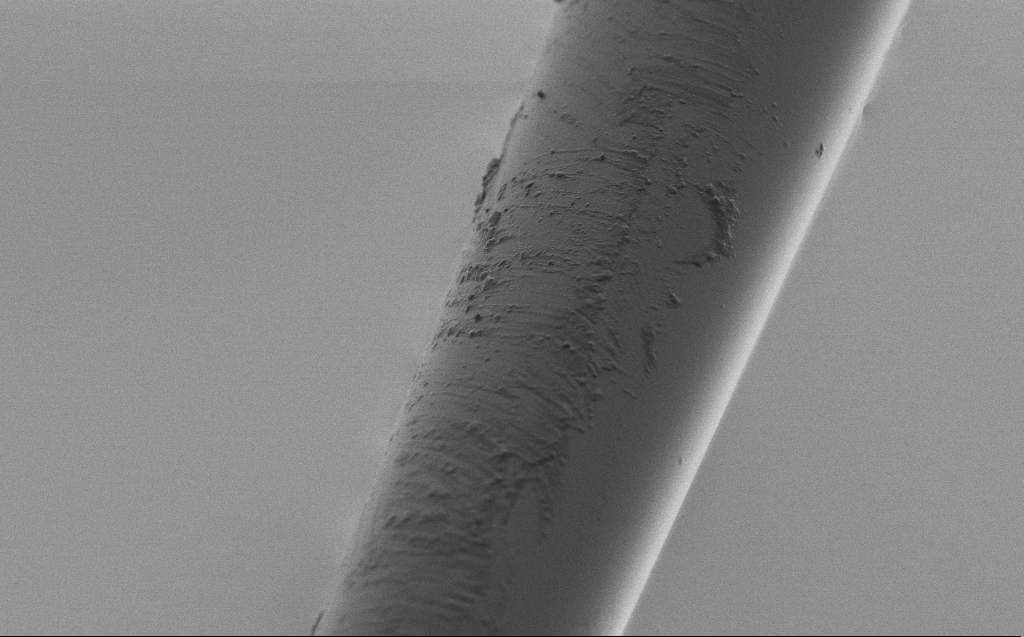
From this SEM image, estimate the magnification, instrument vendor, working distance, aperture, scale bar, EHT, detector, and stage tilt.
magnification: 5 K X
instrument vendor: Zeiss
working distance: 3 mm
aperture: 30 µm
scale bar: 10000 nm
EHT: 2 kV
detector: SE2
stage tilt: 45°